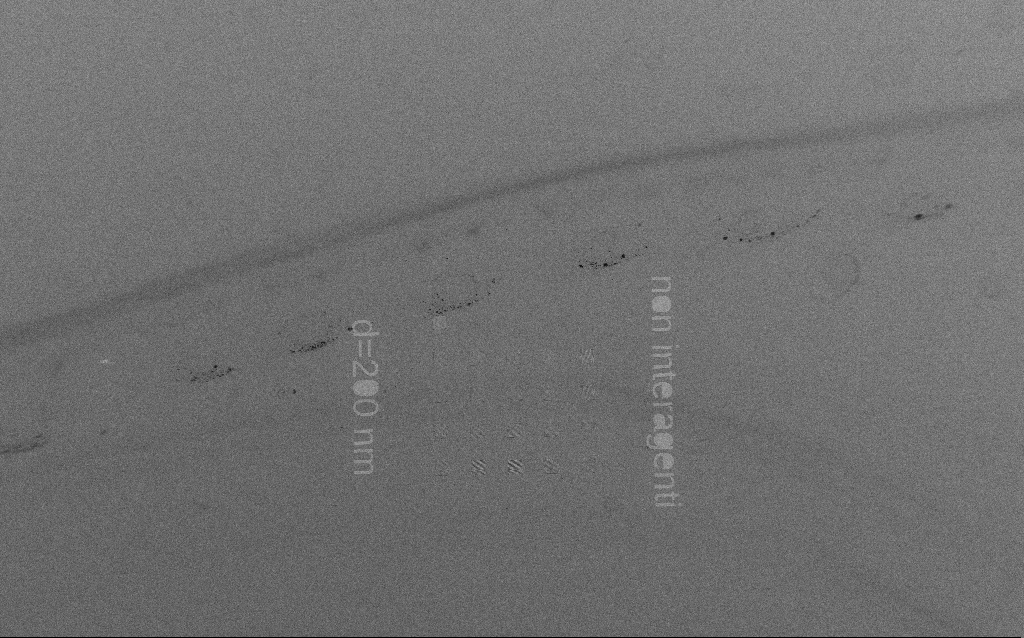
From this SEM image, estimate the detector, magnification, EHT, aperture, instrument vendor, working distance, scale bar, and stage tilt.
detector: SE2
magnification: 0.182 K X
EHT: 1.5 kV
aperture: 30 µm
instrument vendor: Zeiss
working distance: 5.9 mm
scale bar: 100000 nm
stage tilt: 0°